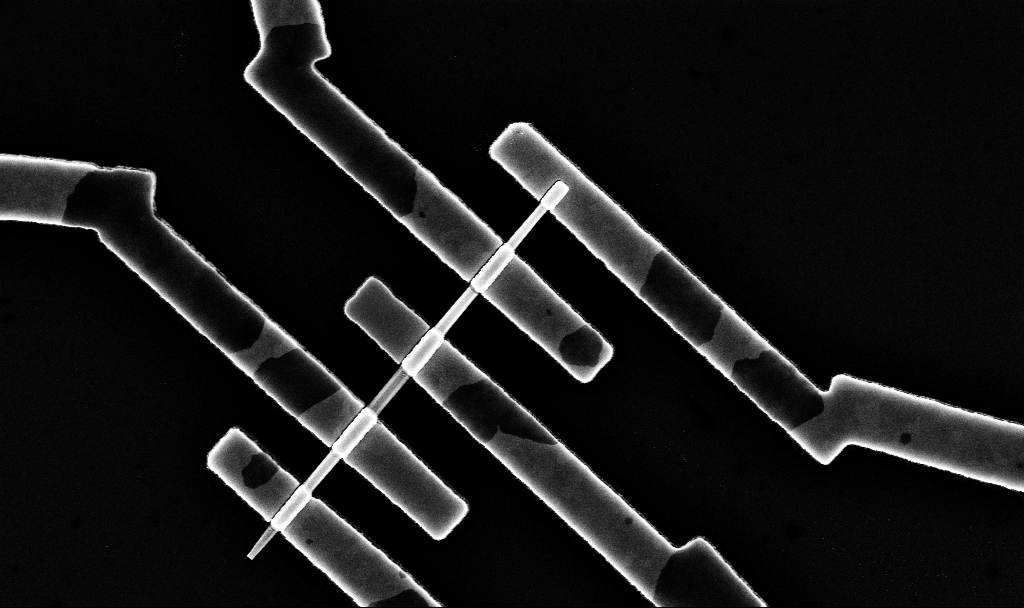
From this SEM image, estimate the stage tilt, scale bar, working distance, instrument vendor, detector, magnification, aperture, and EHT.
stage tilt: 0°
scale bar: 2000 nm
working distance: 6.7 mm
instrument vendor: Zeiss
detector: InLens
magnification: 33.82 K X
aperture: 30 µm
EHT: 10 kV